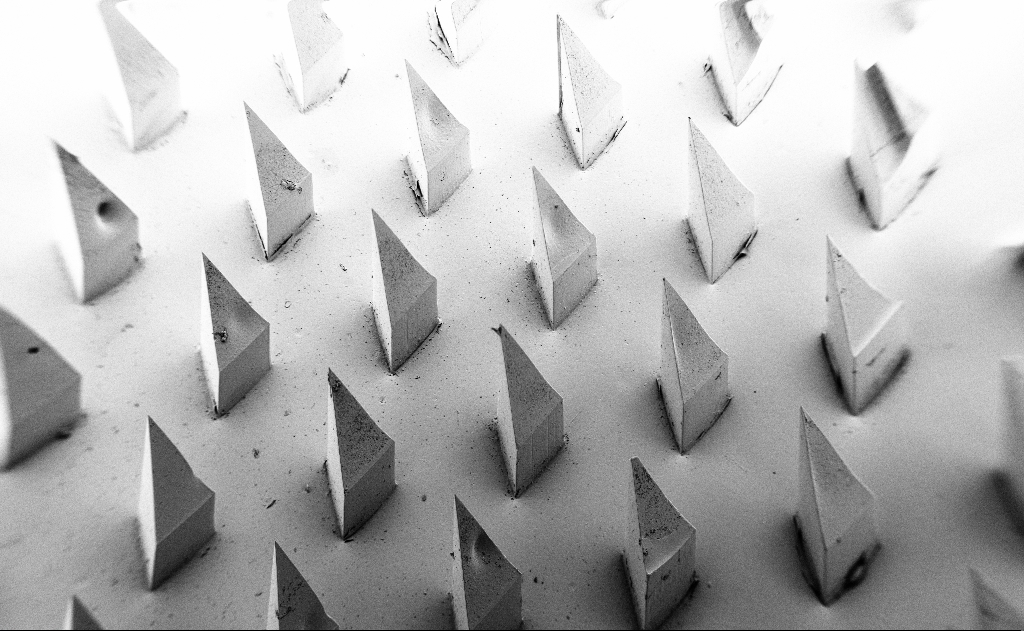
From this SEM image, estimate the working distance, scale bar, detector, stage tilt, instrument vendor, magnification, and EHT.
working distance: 9 mm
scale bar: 1e+06 nm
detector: SE2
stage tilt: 45°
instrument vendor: Zeiss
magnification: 0.067 K X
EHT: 5 kV